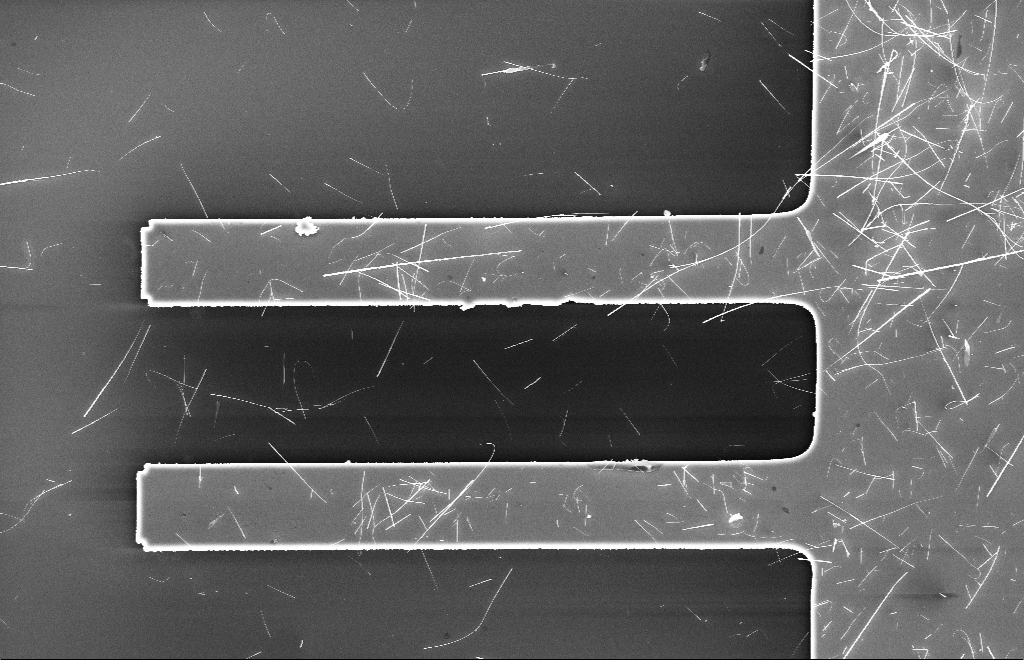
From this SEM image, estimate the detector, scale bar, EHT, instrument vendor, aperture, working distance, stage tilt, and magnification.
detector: InLens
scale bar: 10000 nm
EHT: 10 kV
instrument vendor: Zeiss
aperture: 20 µm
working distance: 6 mm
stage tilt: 0°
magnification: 2.5 K X